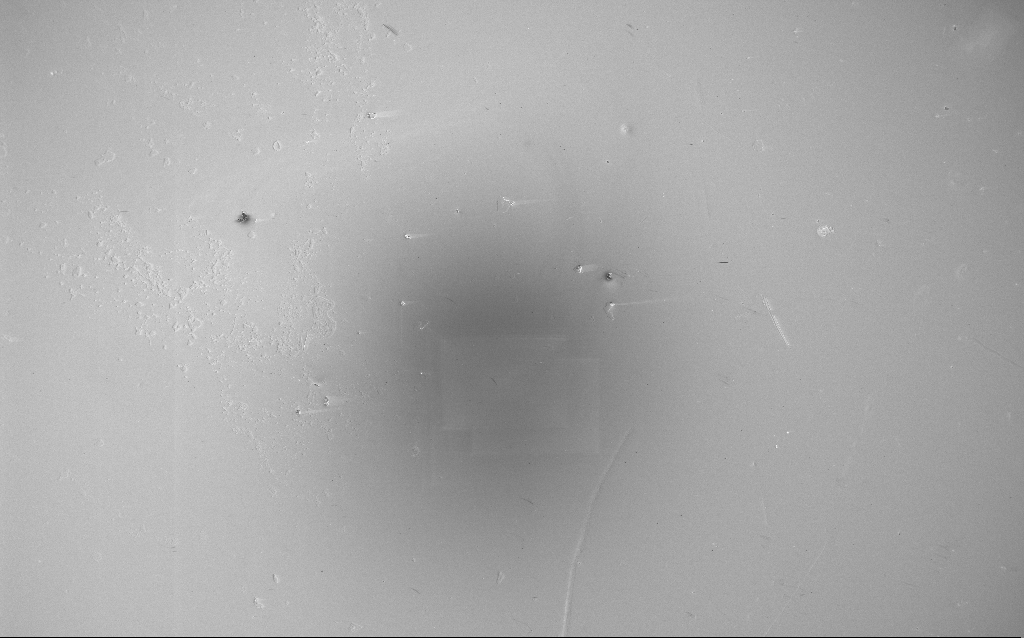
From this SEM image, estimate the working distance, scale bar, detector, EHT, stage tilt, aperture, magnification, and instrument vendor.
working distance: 4 mm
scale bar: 100000 nm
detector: InLens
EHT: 5 kV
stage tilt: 0°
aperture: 30 µm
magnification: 0.149 K X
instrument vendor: Zeiss